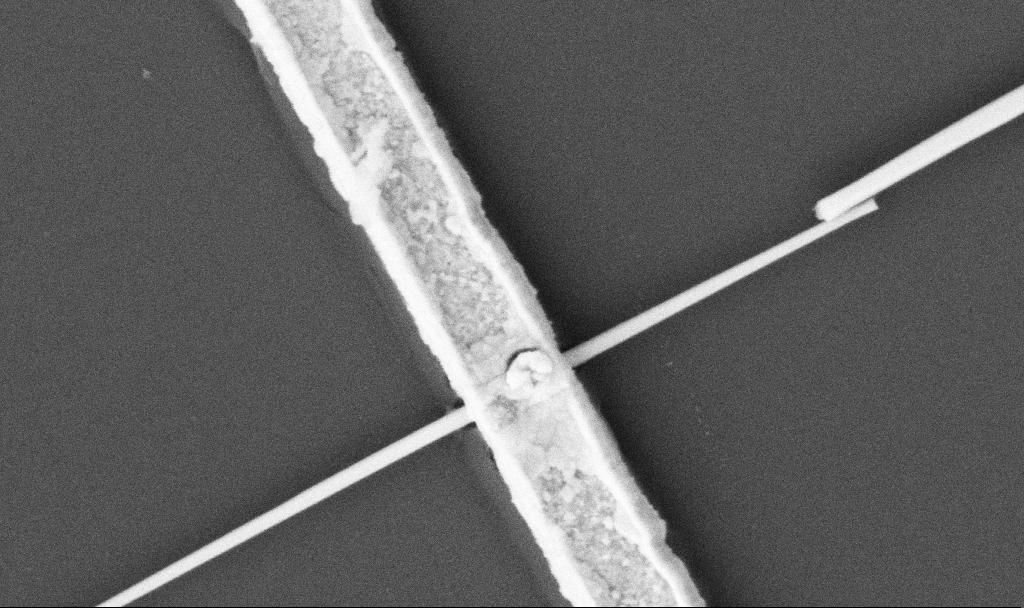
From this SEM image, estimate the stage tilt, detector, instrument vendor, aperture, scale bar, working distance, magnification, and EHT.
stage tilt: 0°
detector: SE2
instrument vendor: Zeiss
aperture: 30 µm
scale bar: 1000 nm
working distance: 10.7 mm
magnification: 60 K X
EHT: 5 kV